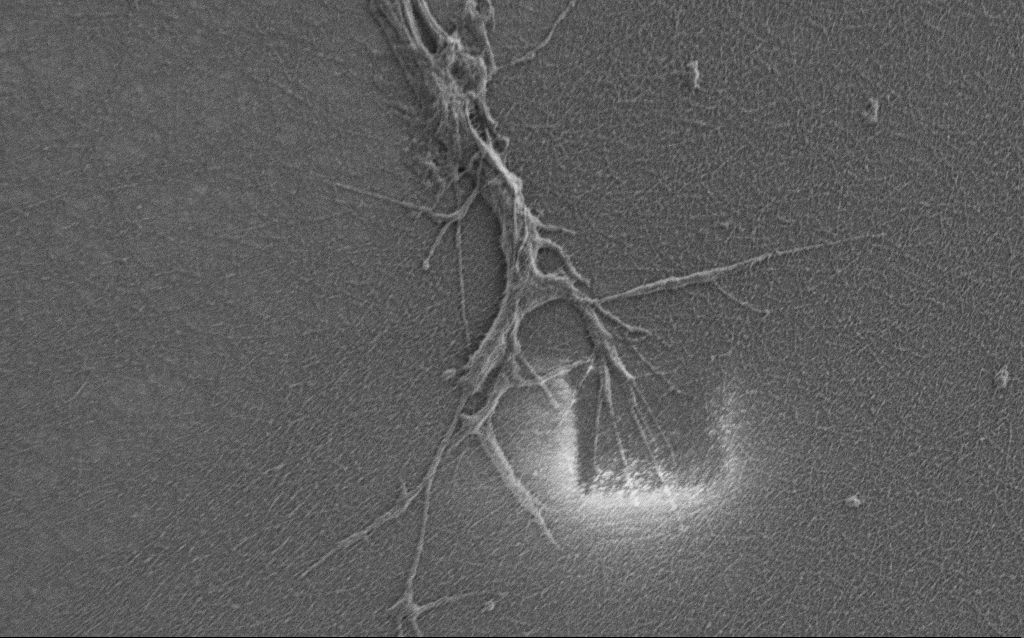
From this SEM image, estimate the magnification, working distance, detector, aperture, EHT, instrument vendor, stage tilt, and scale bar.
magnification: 10 K X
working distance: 6 mm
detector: SE2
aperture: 30 µm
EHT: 0.9 kV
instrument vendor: Zeiss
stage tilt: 0°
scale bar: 2000 nm